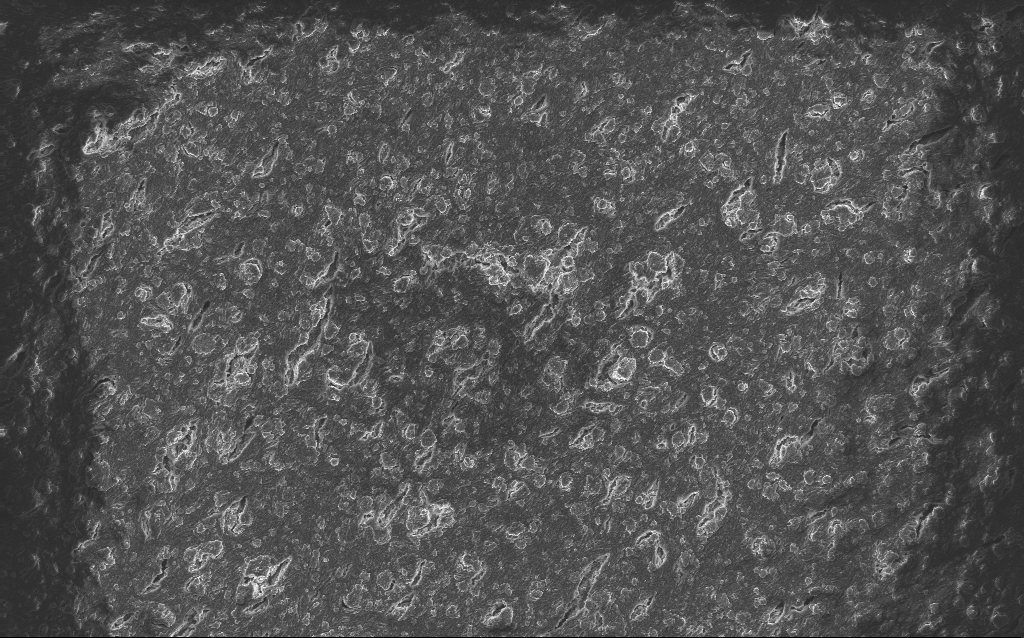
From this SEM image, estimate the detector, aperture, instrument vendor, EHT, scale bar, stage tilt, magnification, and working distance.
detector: InLens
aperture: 30 µm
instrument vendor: Zeiss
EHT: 10 kV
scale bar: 20000 nm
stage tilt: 0°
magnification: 0.792 K X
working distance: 2.6 mm